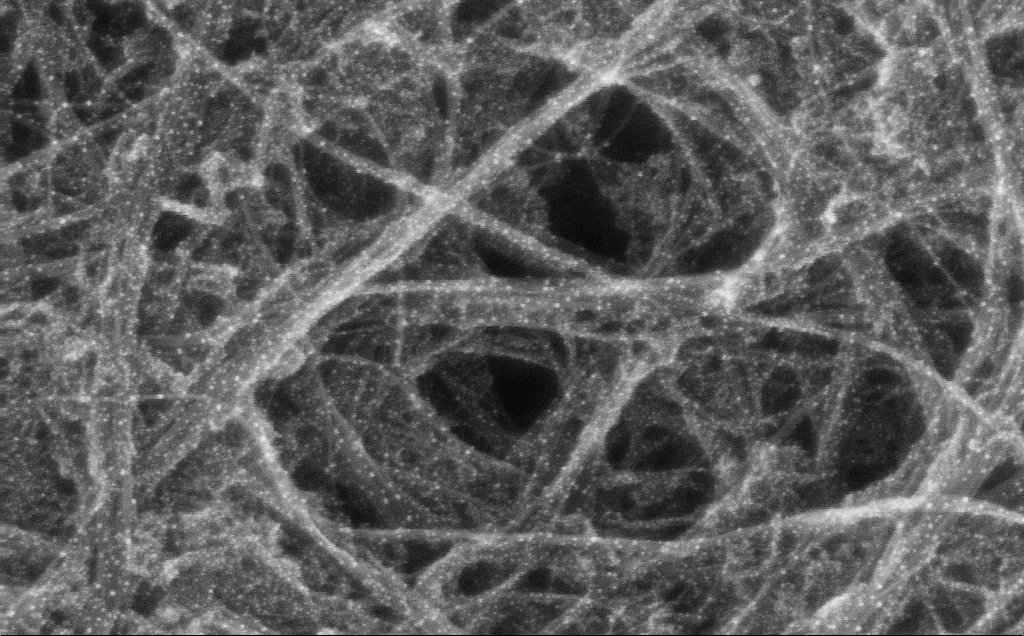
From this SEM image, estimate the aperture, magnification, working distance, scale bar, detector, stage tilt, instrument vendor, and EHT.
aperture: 30 µm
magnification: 251.59 K X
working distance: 3 mm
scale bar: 200 nm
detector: InLens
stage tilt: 0°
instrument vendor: Zeiss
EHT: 10 kV